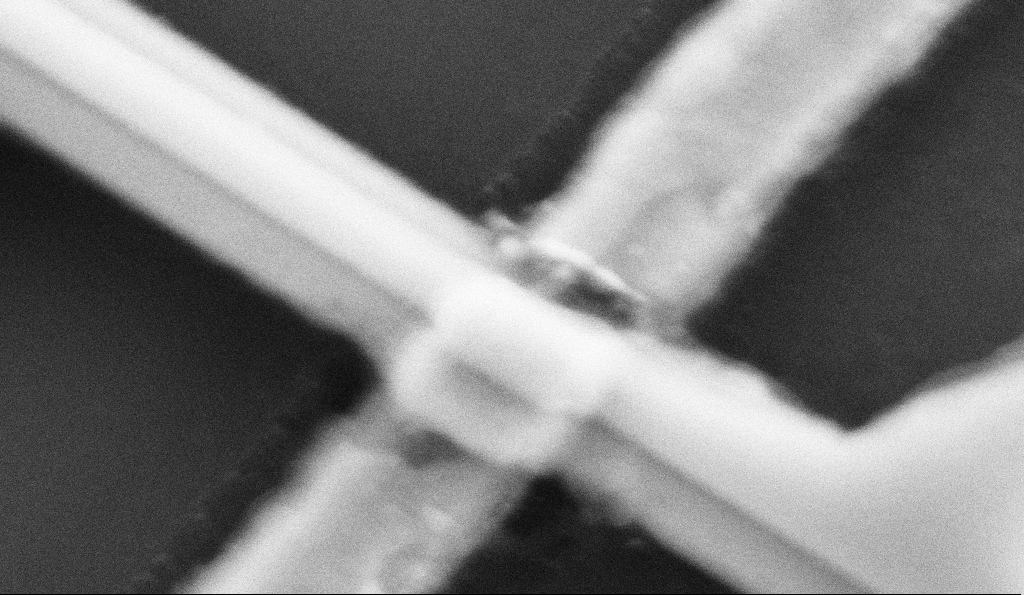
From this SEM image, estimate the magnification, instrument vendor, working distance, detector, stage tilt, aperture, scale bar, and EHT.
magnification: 261.17 K X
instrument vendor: Zeiss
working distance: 8.5 mm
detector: SE2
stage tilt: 0°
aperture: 30 µm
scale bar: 200 nm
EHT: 5 kV